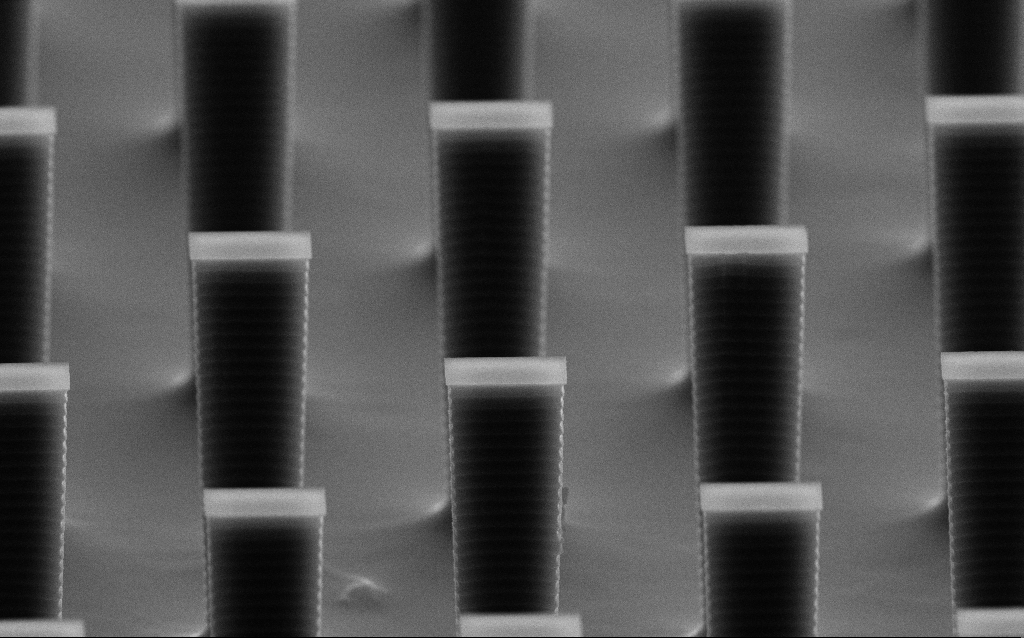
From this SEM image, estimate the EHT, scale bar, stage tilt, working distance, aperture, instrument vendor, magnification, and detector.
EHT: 10 kV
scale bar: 1000 nm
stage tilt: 70°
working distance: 7.5 mm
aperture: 30 µm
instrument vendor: Zeiss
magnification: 15.16 K X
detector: SE2